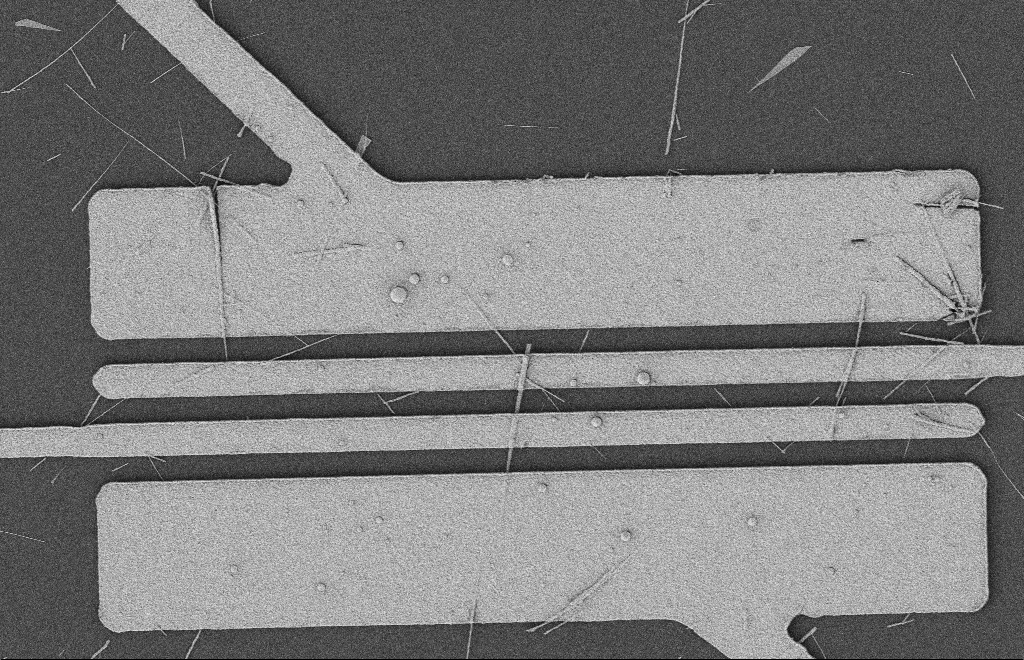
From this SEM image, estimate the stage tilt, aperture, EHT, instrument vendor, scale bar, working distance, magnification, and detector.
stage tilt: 0°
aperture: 20 µm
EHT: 2 kV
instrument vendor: Zeiss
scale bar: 2000 nm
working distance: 12 mm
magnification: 5.33 K X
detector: SE2